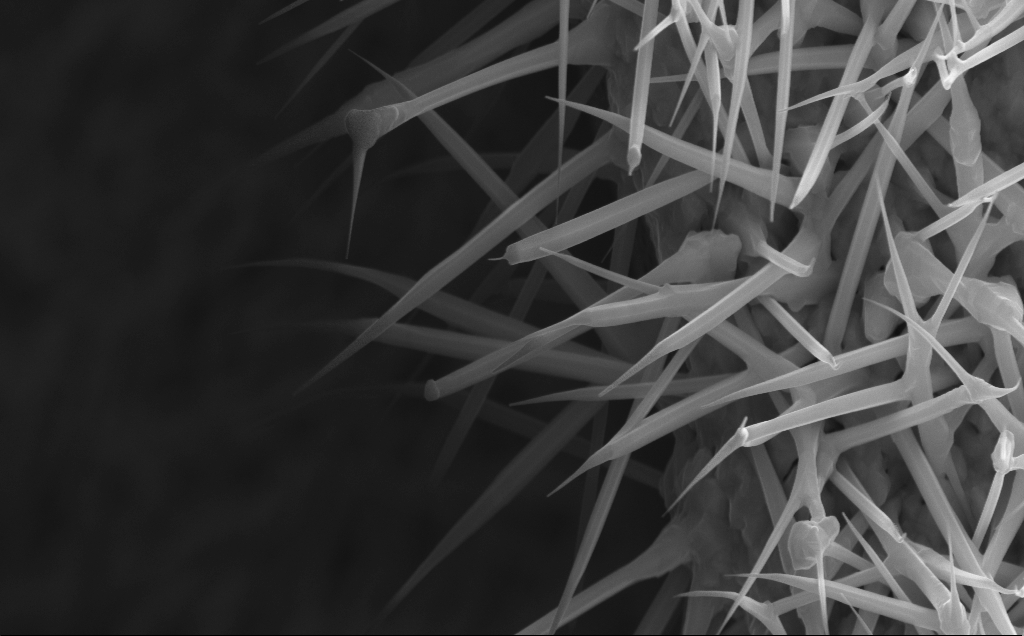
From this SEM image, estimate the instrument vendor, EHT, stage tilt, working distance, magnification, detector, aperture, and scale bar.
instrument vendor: Zeiss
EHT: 10 kV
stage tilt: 0°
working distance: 7 mm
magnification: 44.2 K X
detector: InLens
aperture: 30 µm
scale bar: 1000 nm